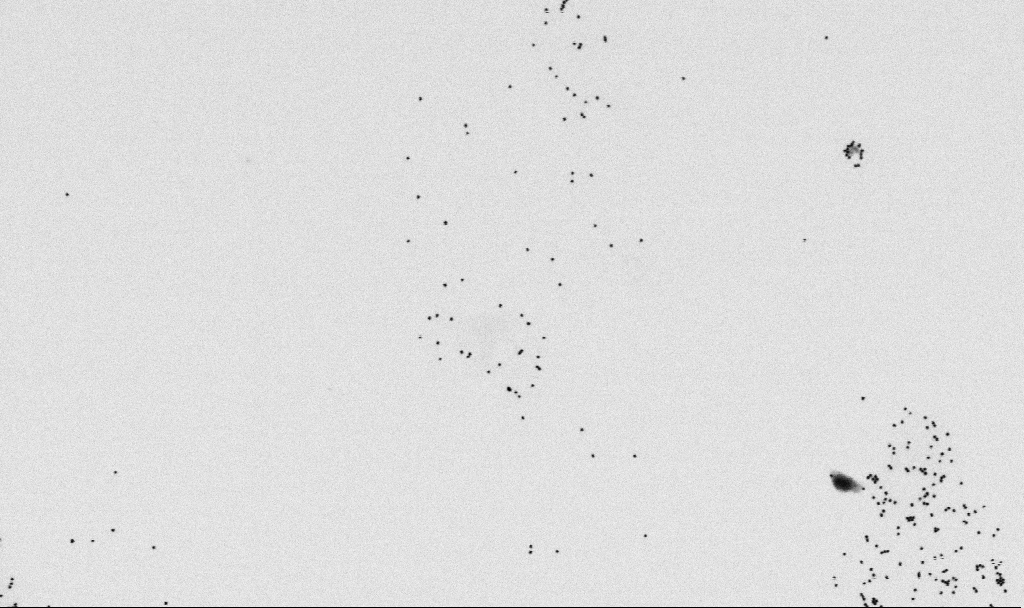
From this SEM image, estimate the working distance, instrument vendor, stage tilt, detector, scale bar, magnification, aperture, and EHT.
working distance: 6.5 mm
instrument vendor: Zeiss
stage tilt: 0°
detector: SE2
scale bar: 1000 nm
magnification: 54.45 K X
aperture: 30 µm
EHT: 2 kV